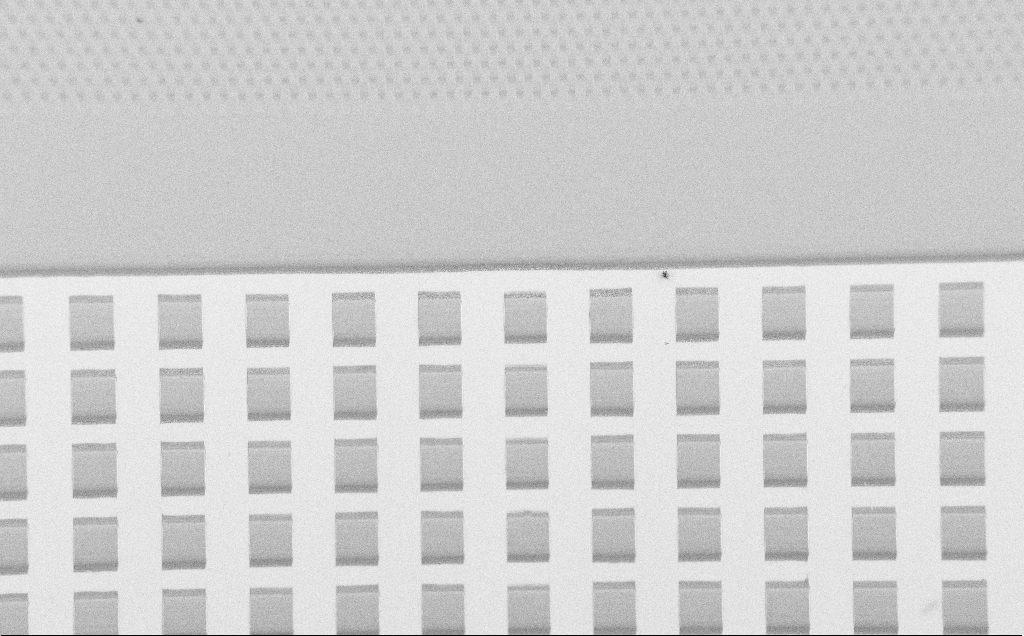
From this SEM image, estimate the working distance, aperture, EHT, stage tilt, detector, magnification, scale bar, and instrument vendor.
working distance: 6 mm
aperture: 30 µm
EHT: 1.5 kV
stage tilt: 30°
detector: SE2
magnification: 0.317 K X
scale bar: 200000 nm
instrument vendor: Zeiss